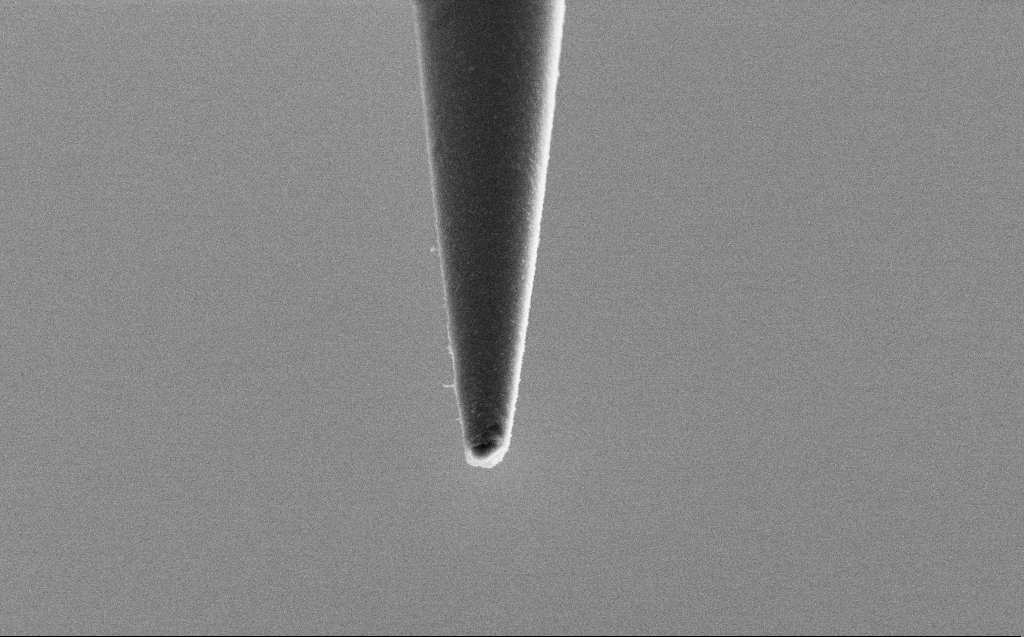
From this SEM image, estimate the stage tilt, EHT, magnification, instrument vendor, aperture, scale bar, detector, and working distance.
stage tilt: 45°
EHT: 4 kV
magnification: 25 K X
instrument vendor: Zeiss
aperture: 30 µm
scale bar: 2000 nm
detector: SE2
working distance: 4 mm